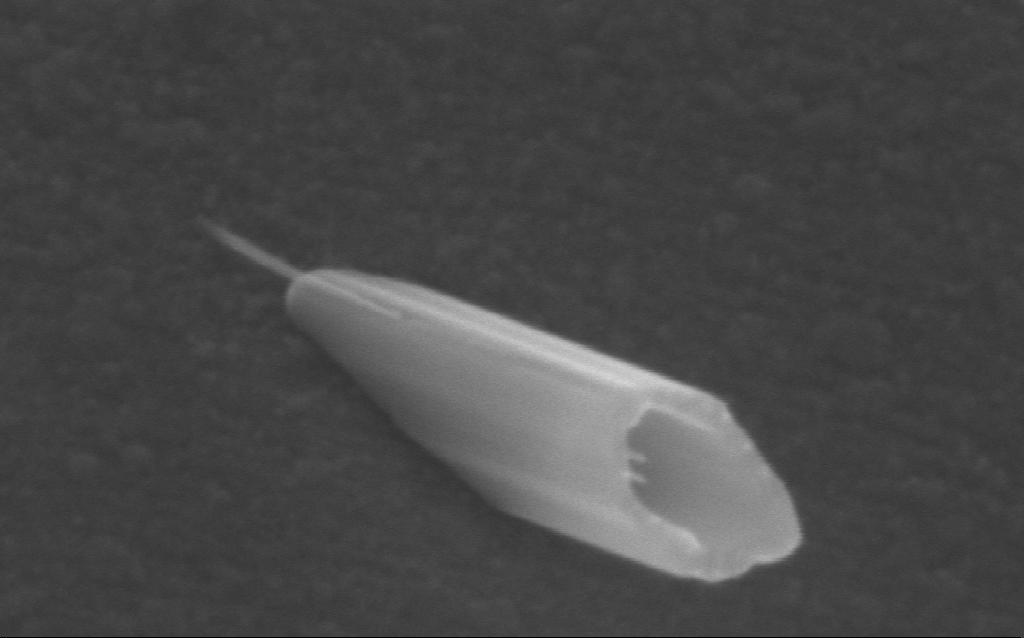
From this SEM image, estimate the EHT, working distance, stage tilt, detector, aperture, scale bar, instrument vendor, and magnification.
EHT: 5 kV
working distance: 3 mm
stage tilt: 23.6°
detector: InLens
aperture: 30 µm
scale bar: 100 nm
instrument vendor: Zeiss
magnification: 554.25 K X